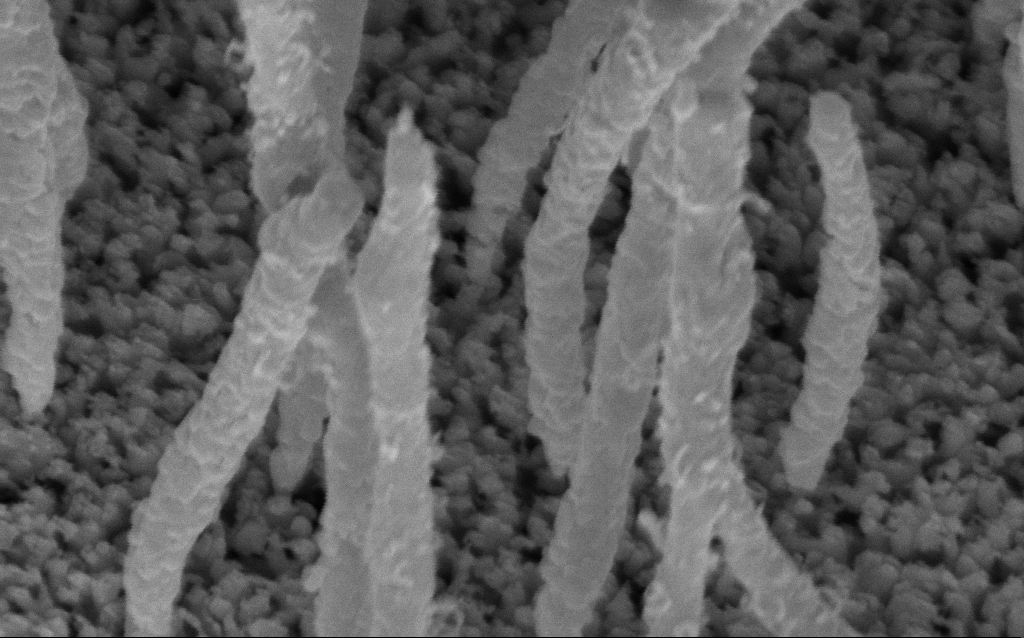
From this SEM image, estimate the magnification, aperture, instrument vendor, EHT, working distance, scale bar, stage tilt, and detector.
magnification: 200 K X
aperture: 30 µm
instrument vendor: Zeiss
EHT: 5 kV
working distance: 8.2 mm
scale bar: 100 nm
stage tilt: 45°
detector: InLens